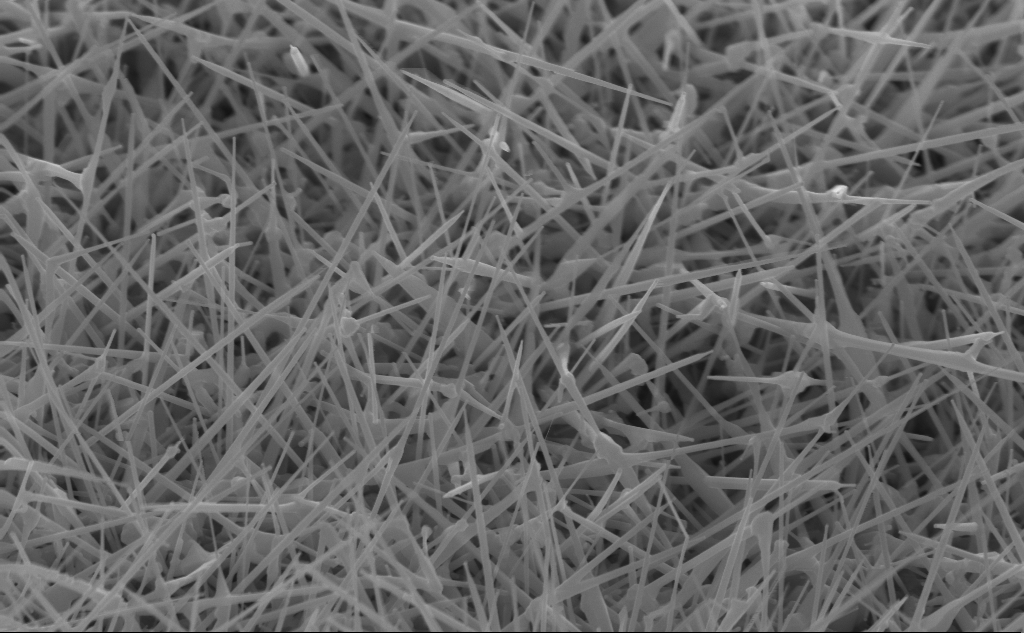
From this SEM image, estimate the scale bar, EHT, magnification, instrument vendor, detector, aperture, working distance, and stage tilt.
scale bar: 1000 nm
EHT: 10 kV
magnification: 40 K X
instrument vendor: Zeiss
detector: InLens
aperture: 30 µm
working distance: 4 mm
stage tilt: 45°